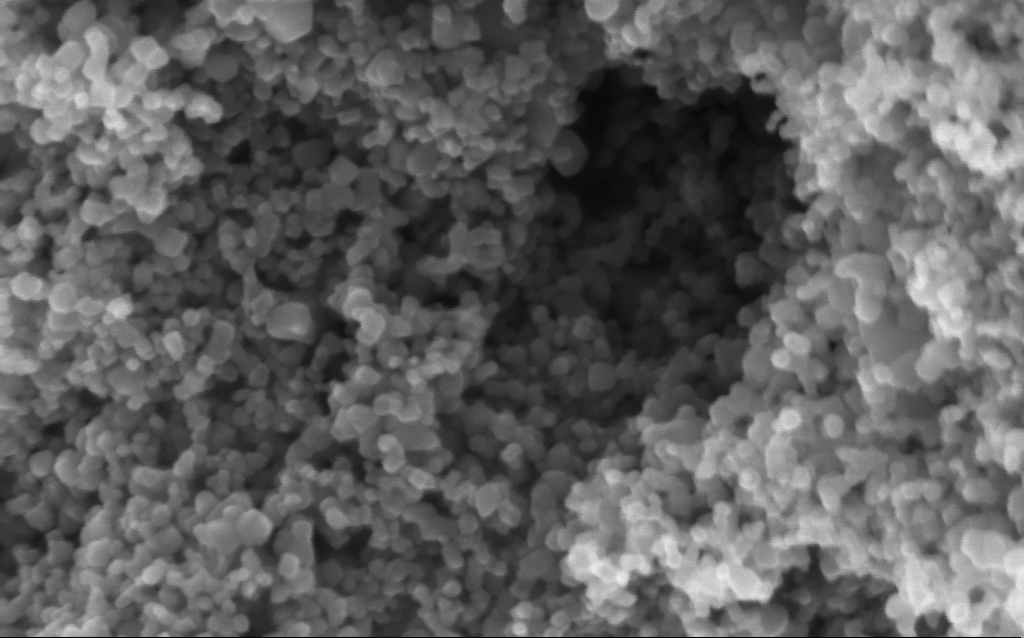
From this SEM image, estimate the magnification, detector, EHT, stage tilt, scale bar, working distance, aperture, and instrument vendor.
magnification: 282.07 K X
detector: InLens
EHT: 5 kV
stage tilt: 0°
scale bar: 200 nm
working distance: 4.2 mm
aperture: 30 µm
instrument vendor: Zeiss